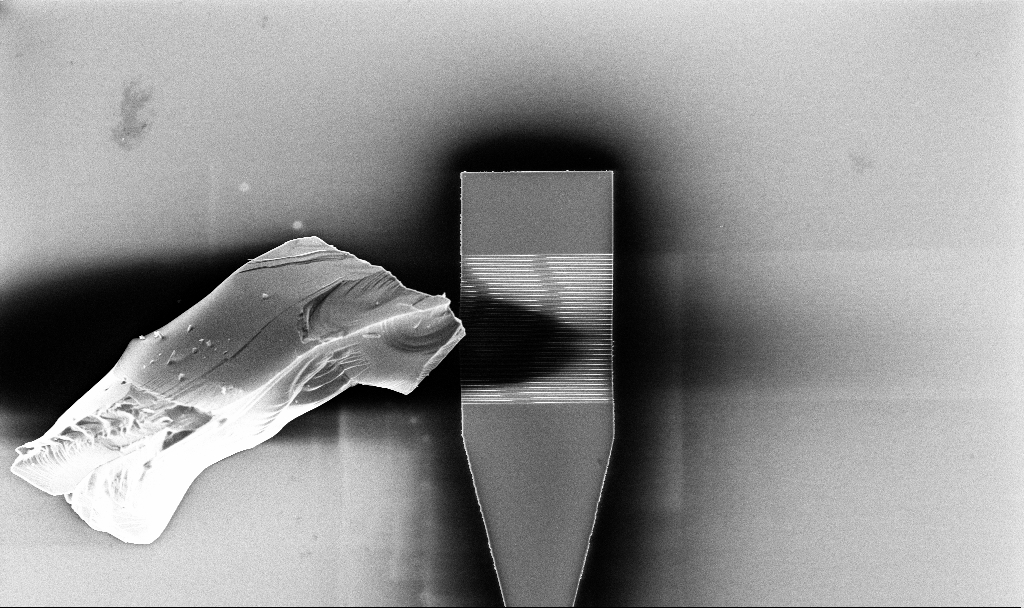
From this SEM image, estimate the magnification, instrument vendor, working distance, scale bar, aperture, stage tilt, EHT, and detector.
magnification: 2.83 K X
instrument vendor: Zeiss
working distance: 5.2 mm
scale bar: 10000 nm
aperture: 30 µm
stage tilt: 0°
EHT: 5 kV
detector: InLens